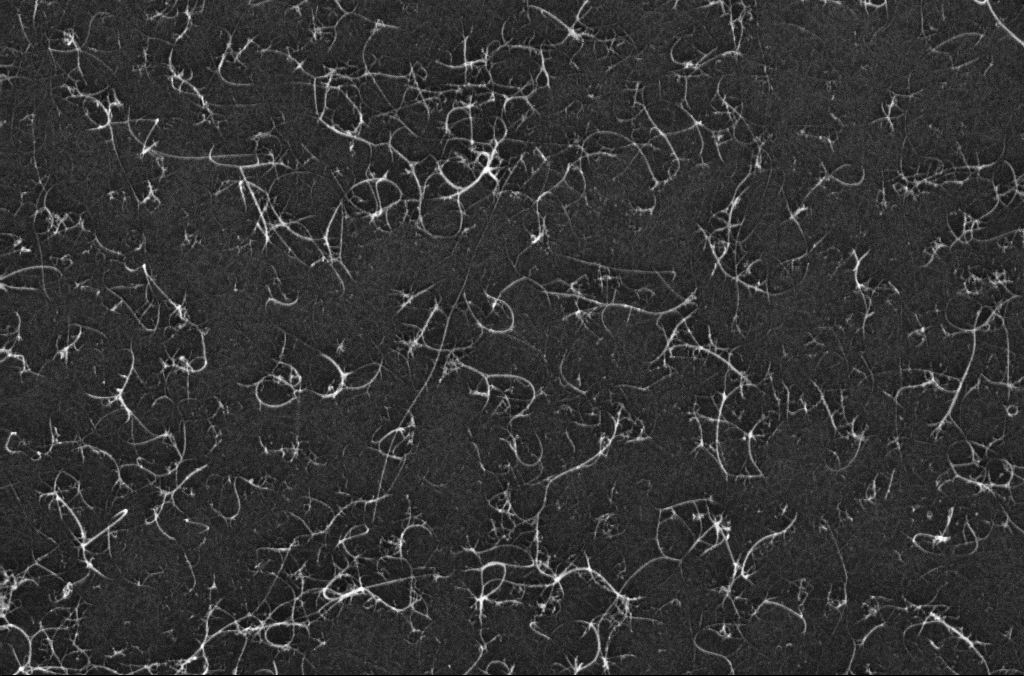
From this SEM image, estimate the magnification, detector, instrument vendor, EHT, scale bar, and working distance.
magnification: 83.75 K X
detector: InLens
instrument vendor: Zeiss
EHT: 10 kV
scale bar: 200 nm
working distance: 3.3 mm